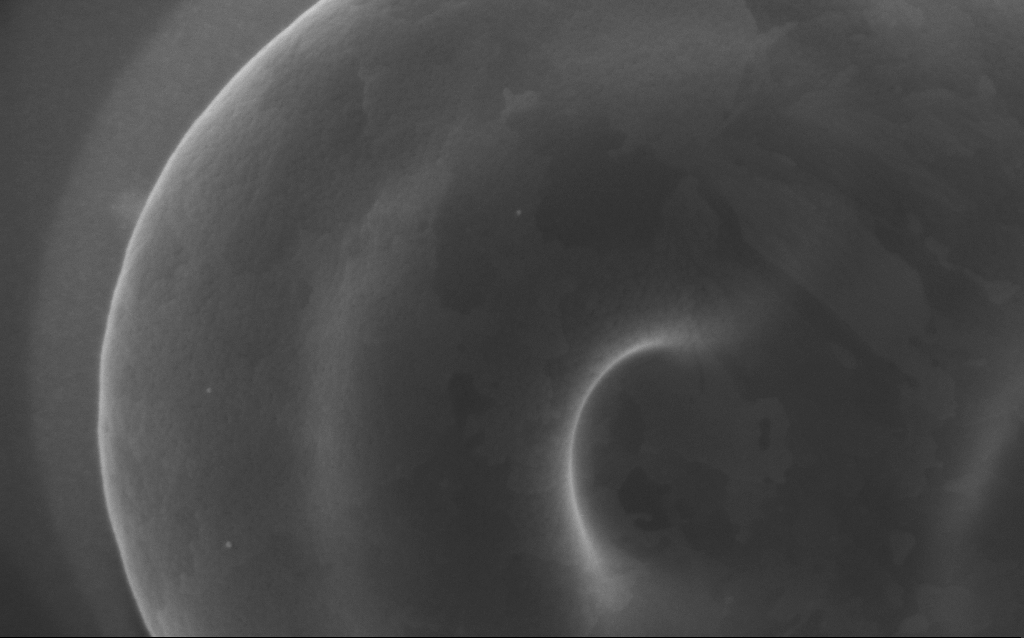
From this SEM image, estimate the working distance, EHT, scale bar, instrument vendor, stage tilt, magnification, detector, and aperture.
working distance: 3 mm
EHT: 5 kV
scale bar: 200 nm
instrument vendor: Zeiss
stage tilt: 0°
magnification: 107.11 K X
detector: InLens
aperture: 30 µm